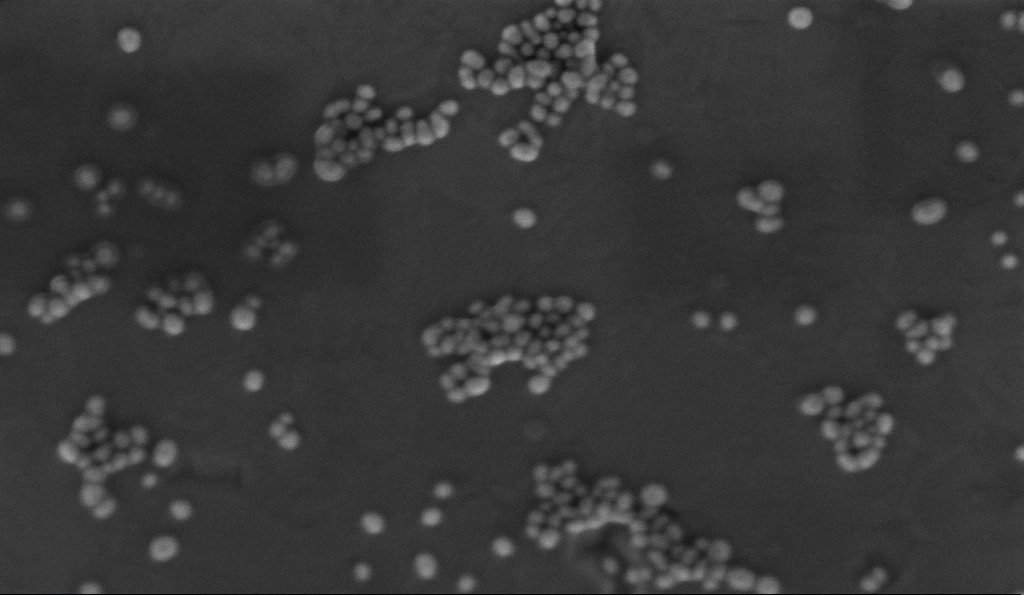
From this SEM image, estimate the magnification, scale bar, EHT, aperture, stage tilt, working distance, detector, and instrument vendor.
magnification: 250 K X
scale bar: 100 nm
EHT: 2 kV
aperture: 30 µm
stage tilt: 0°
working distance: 2.3 mm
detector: InLens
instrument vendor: Zeiss